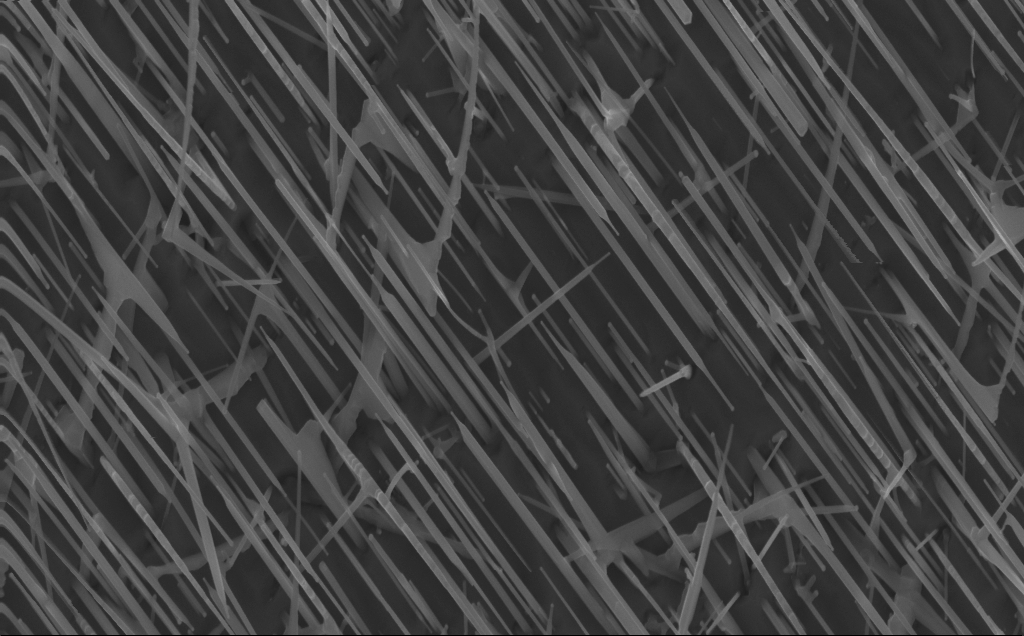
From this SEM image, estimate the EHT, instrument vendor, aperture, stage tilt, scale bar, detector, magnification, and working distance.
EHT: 10 kV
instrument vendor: Zeiss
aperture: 30 µm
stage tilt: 0°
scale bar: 1000 nm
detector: InLens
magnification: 40 K X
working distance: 4 mm